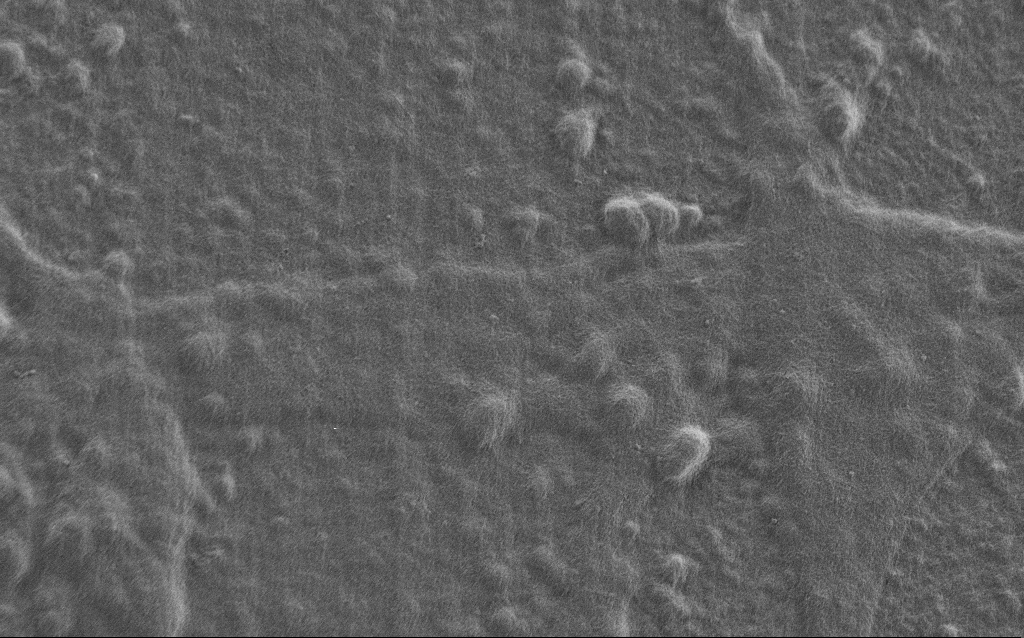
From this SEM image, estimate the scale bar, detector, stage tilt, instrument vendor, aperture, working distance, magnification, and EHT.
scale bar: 10000 nm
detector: SE2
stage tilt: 0°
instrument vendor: Zeiss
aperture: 30 µm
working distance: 7 mm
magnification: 5 K X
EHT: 0.9 kV